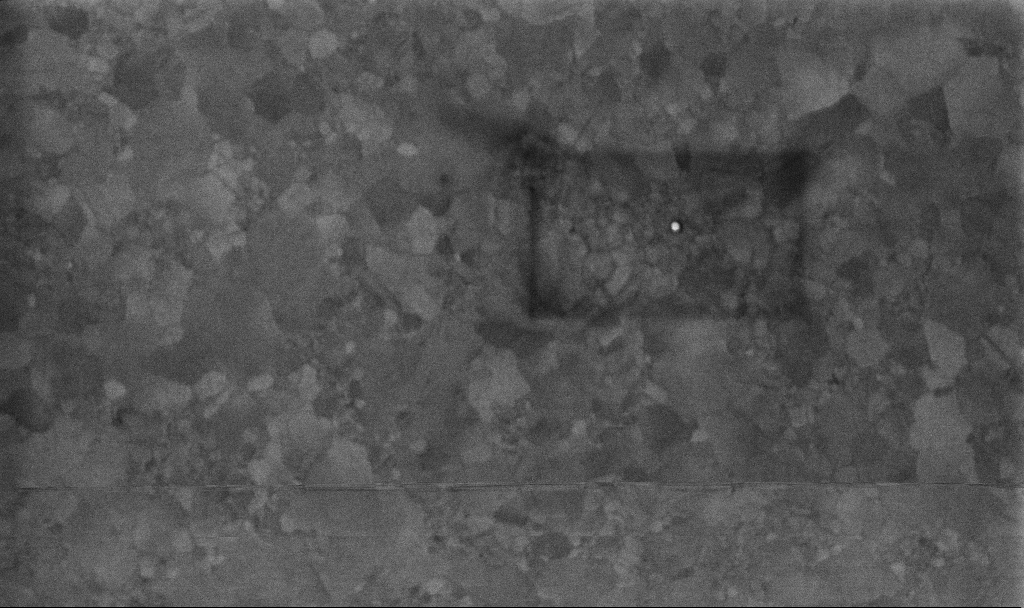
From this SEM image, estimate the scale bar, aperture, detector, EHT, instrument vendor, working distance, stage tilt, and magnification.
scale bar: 200 nm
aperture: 30 µm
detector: InLens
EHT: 10 kV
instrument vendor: Zeiss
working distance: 3.4 mm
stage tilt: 0°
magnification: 100 K X